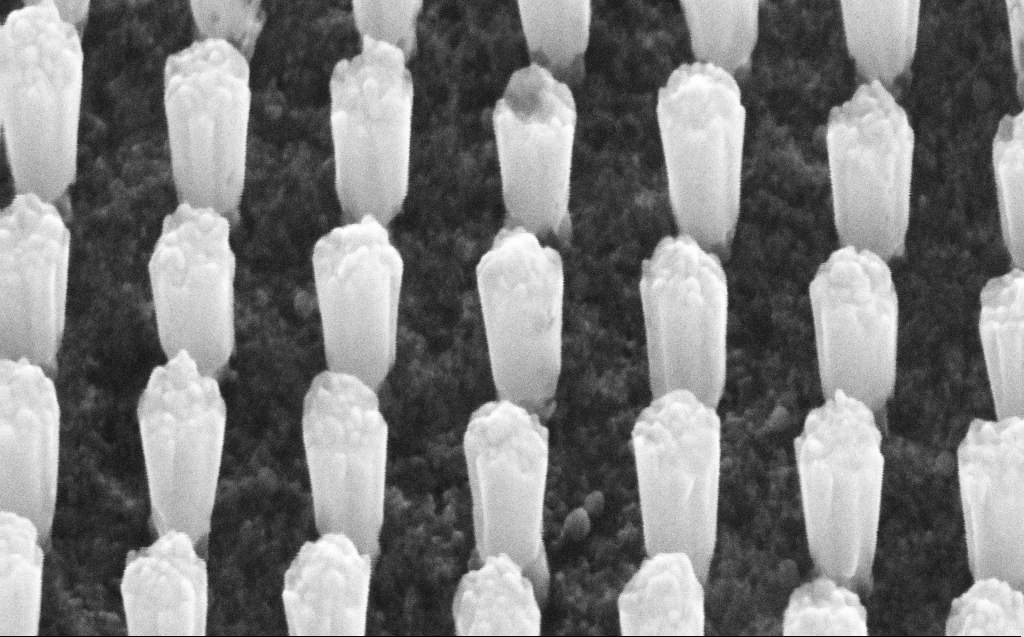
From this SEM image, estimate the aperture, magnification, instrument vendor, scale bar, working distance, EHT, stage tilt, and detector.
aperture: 30 µm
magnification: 310.54 K X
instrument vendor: Zeiss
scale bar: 200 nm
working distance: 5 mm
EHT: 30 kV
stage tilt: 45°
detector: SE2